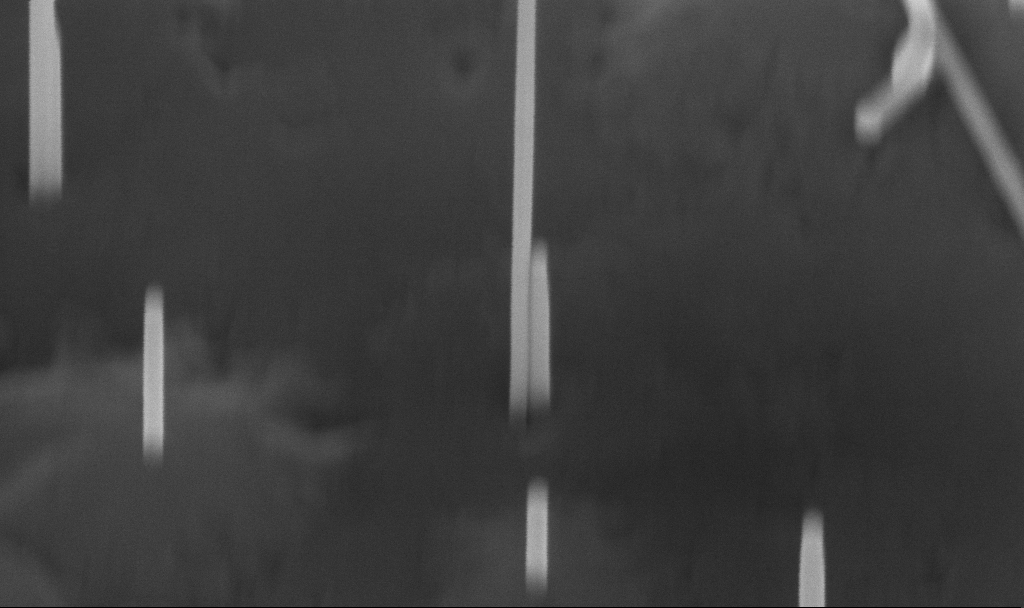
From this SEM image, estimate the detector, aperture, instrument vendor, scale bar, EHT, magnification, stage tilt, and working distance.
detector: InLens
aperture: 30 µm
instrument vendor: Zeiss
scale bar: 200 nm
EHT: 10 kV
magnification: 240.4 K X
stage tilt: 45°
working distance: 5.6 mm